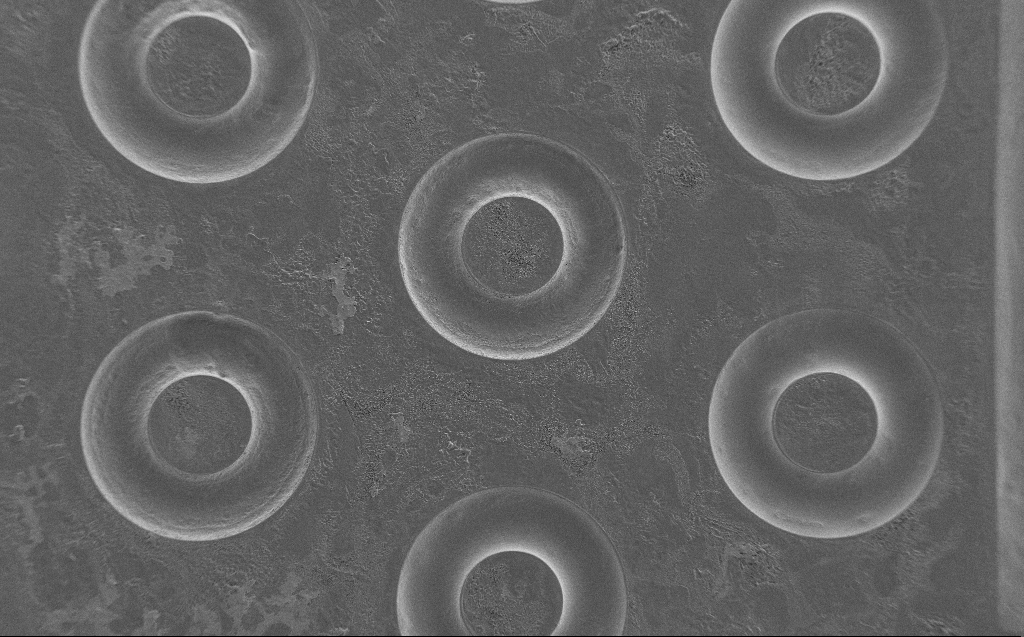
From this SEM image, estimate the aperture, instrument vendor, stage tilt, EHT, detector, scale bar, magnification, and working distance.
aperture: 30 µm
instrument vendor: Zeiss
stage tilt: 0°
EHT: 2 kV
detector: SE2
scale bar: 100000 nm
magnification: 0.234 K X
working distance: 7 mm